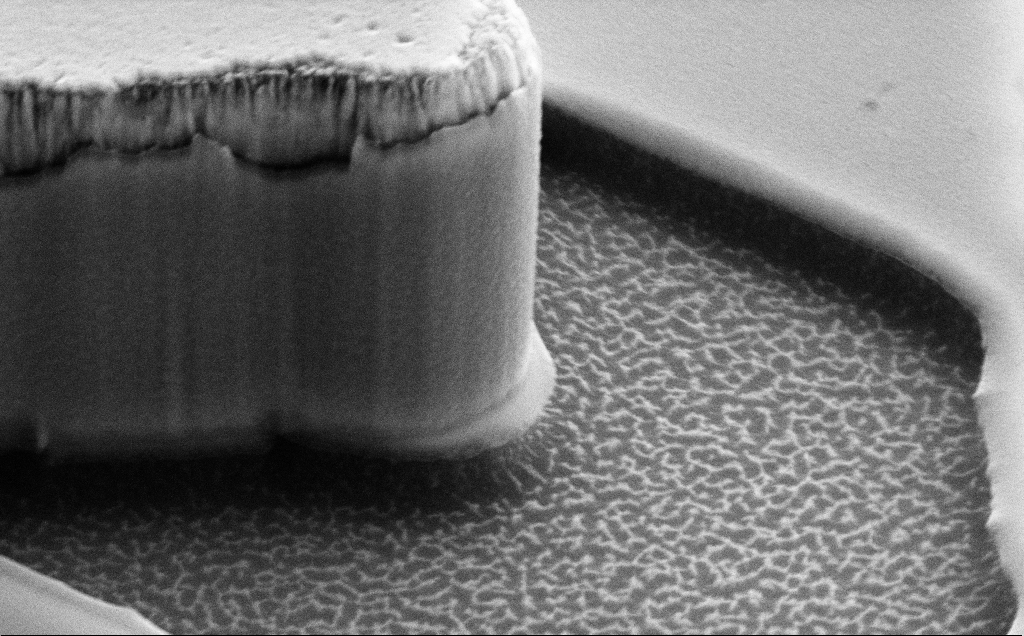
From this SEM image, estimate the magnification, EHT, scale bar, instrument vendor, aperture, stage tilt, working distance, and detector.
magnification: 33.34 K X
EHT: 10 kV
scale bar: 2000 nm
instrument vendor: Zeiss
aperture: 30 µm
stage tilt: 55.6°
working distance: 10 mm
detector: SE2